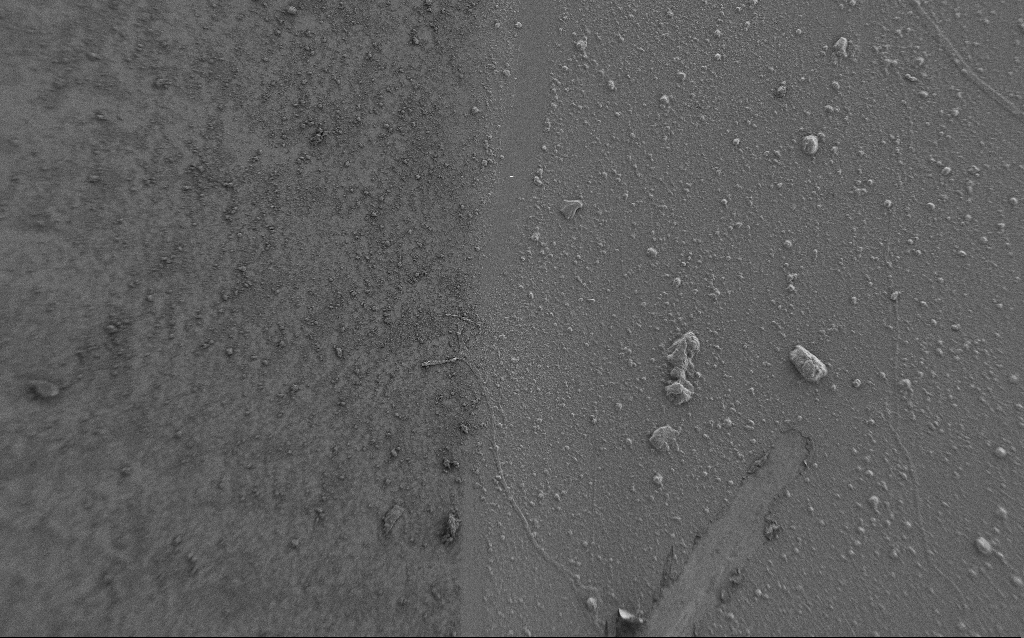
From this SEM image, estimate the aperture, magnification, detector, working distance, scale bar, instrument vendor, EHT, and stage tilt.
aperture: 30 µm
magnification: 0.5 K X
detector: SE2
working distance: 7 mm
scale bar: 100000 nm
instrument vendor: Zeiss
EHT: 0.9 kV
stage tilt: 0°